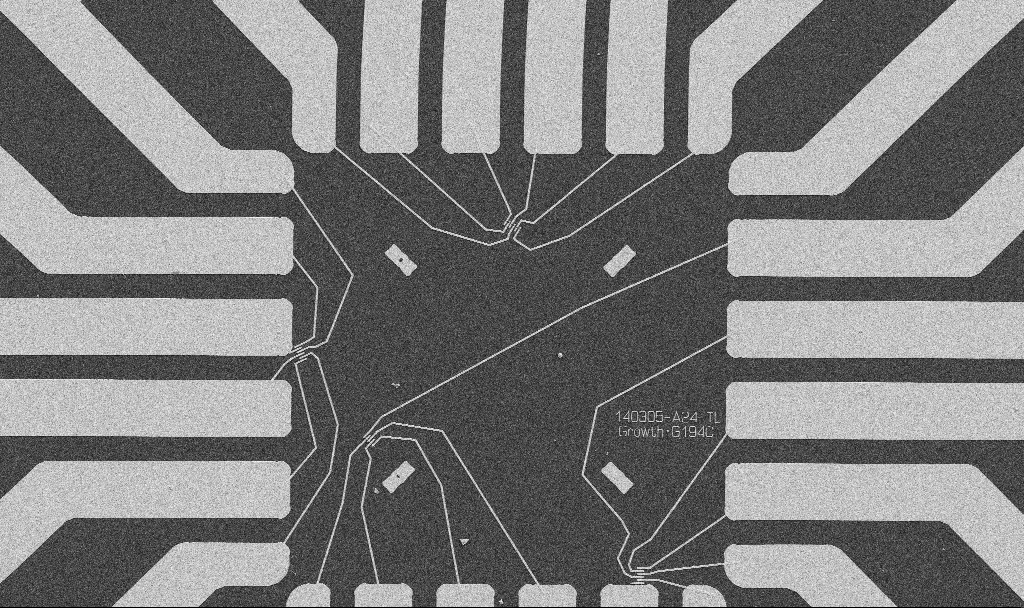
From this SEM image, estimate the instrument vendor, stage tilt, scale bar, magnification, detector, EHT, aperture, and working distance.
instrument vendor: Zeiss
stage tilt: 0°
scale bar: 20000 nm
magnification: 1 K X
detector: SE2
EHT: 5 kV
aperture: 30 µm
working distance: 10.7 mm